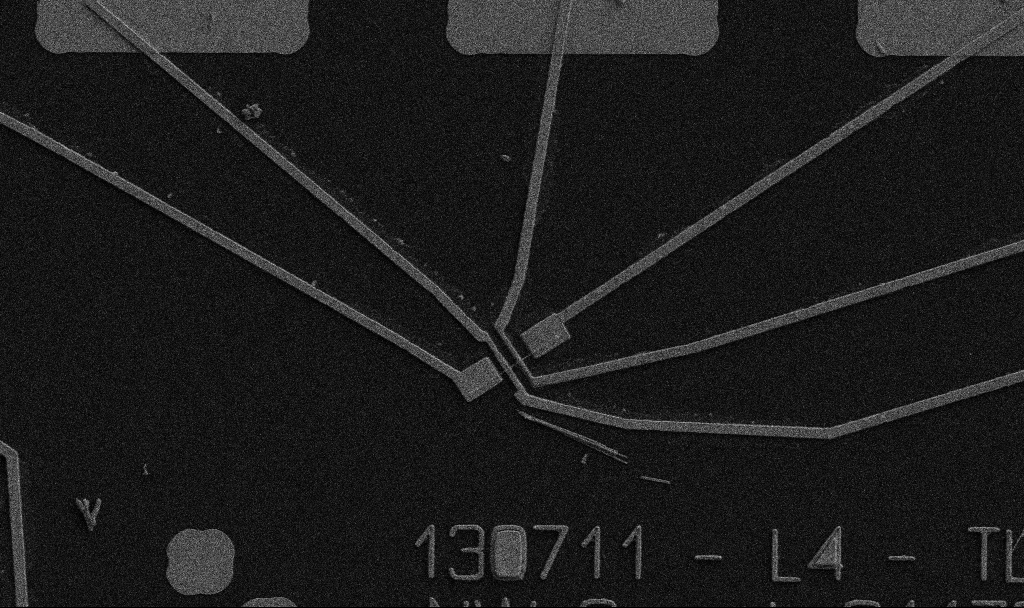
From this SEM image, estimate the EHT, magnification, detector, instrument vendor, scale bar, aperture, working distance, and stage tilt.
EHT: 5 kV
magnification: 5 K X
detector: SE2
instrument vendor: Zeiss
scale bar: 10000 nm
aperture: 30 µm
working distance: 10.7 mm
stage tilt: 0°